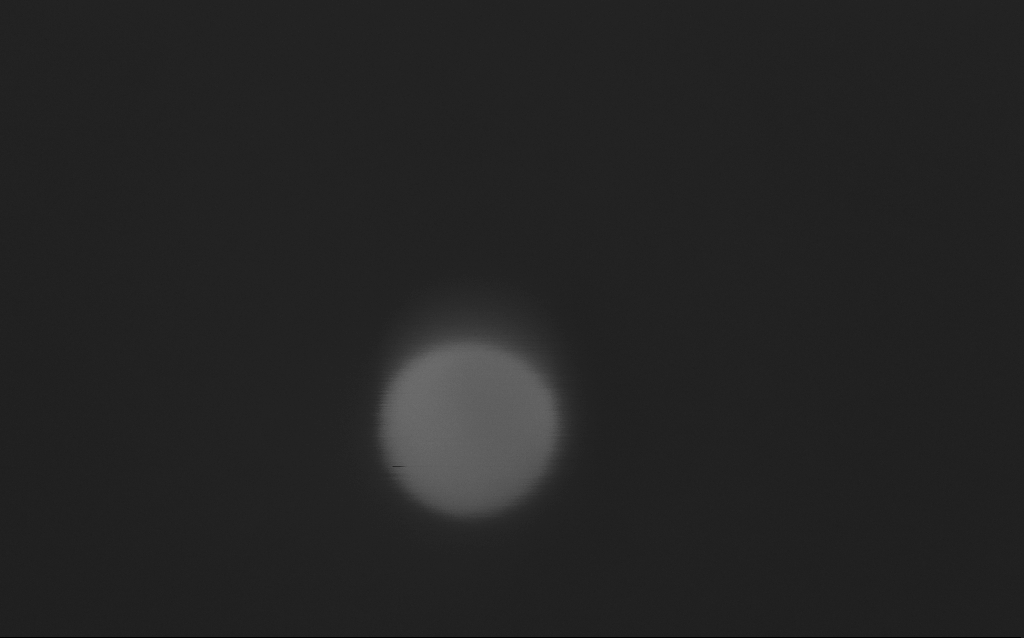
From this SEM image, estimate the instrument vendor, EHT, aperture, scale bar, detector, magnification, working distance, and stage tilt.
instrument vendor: Zeiss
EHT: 5 kV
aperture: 30 µm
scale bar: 20 nm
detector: InLens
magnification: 1005.26 K X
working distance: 3 mm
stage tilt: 0°